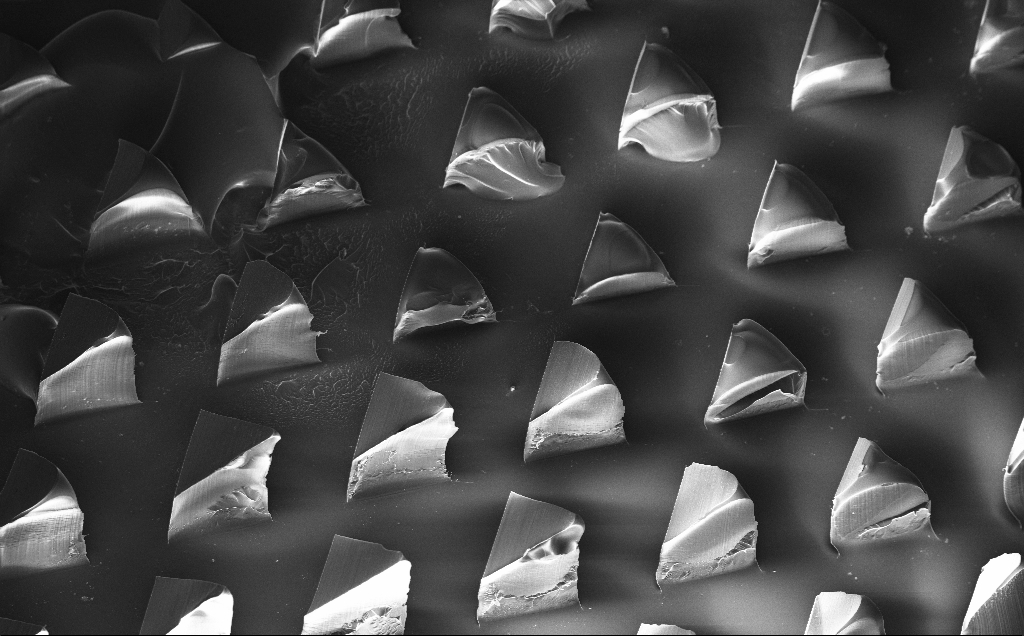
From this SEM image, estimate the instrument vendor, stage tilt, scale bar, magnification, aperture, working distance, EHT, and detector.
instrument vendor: Zeiss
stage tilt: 20°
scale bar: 100000 nm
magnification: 0.148 K X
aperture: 30 µm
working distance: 8 mm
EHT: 10 kV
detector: InLens